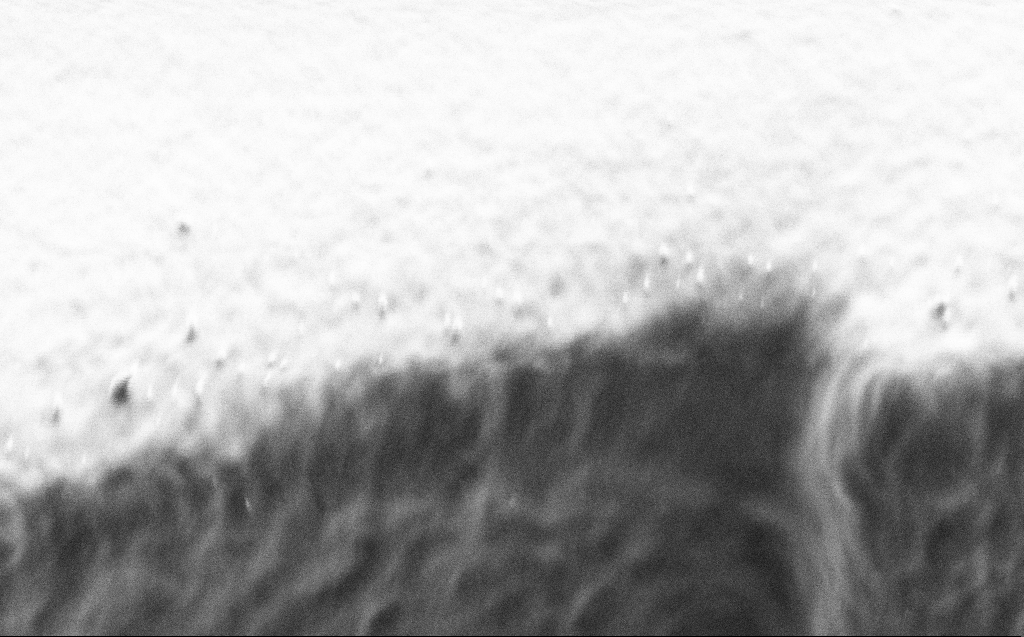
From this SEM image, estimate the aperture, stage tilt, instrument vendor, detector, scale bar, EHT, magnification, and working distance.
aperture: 30 µm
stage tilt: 44.6°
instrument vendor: Zeiss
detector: SE2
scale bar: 1000 nm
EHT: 1 kV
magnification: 39.1 K X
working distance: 5 mm